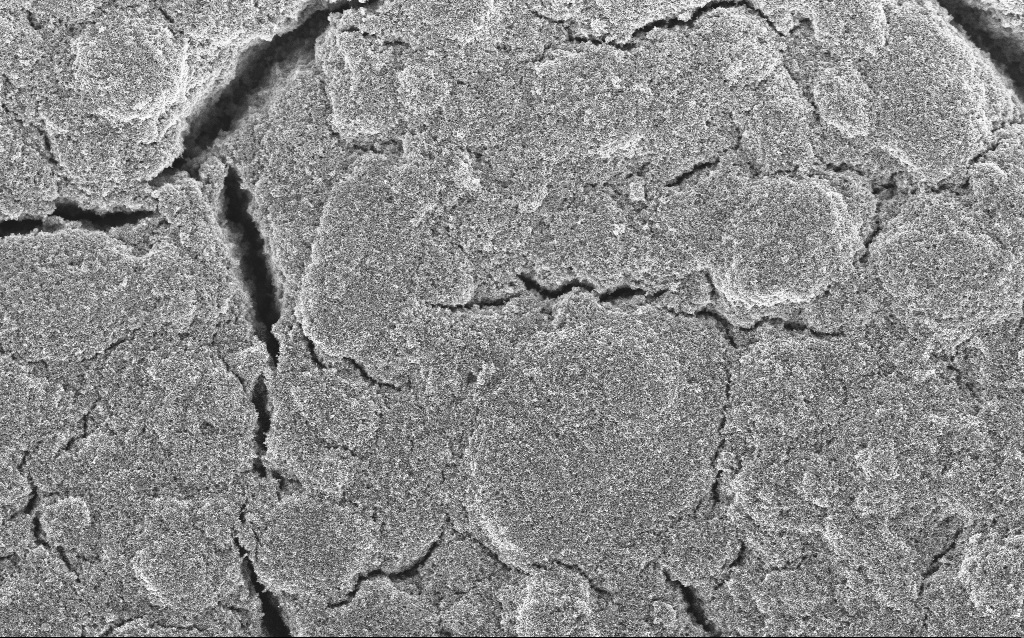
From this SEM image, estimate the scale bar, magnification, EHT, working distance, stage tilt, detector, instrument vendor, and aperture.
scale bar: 10000 nm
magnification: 6.61 K X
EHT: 5 kV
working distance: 4.2 mm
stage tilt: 0°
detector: InLens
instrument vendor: Zeiss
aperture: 30 µm